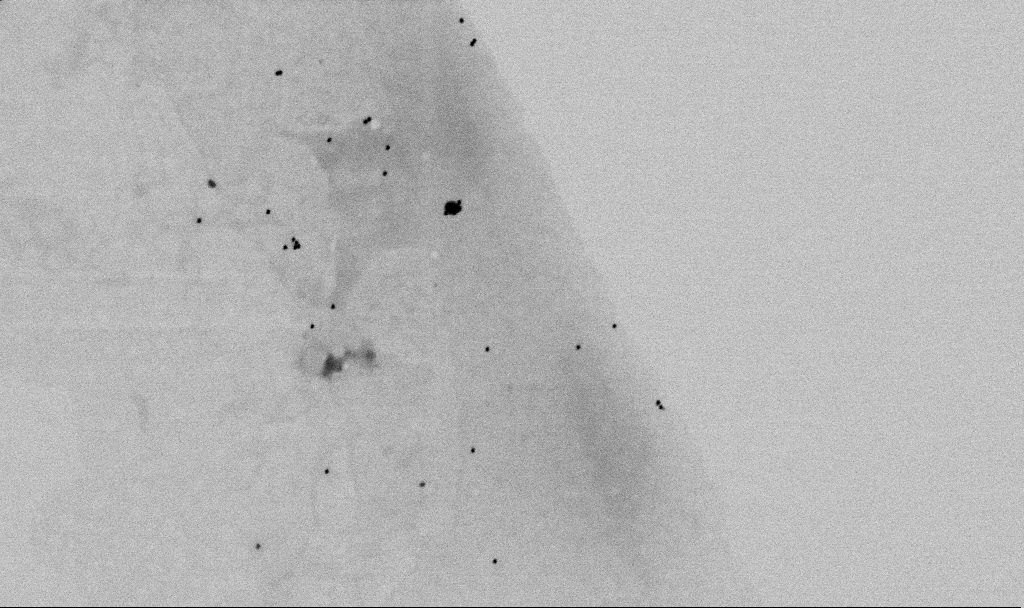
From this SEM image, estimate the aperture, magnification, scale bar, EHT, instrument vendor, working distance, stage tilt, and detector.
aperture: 30 µm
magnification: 60 K X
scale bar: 1000 nm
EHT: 2 kV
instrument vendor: Zeiss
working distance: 5.5 mm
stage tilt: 0°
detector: SE2